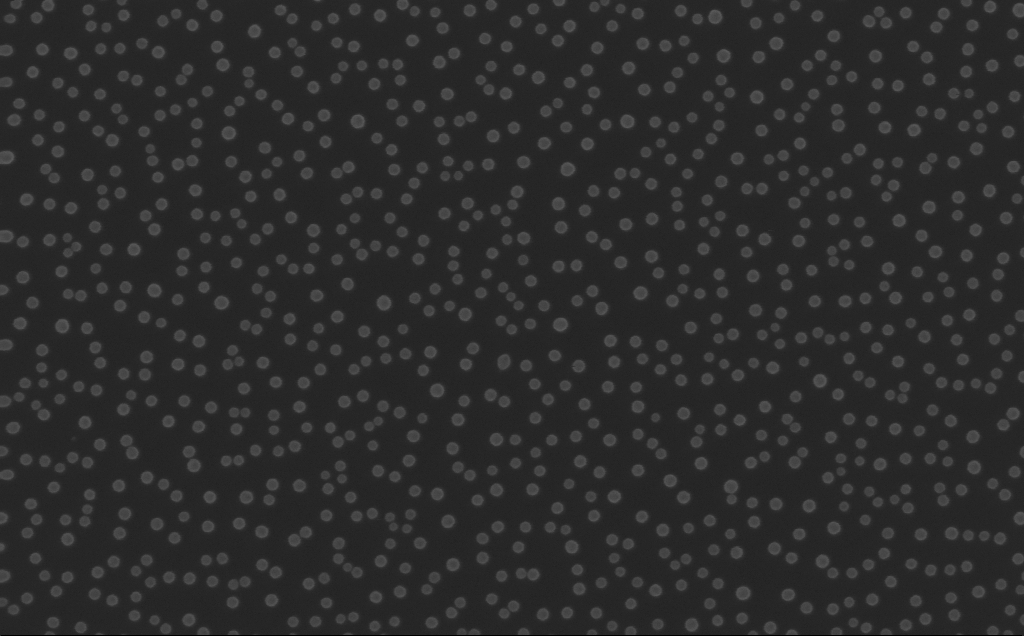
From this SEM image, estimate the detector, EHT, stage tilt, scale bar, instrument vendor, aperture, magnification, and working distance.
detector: InLens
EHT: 10 kV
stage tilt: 0°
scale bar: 100 nm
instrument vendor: Zeiss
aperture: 30 µm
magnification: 150 K X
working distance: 4 mm